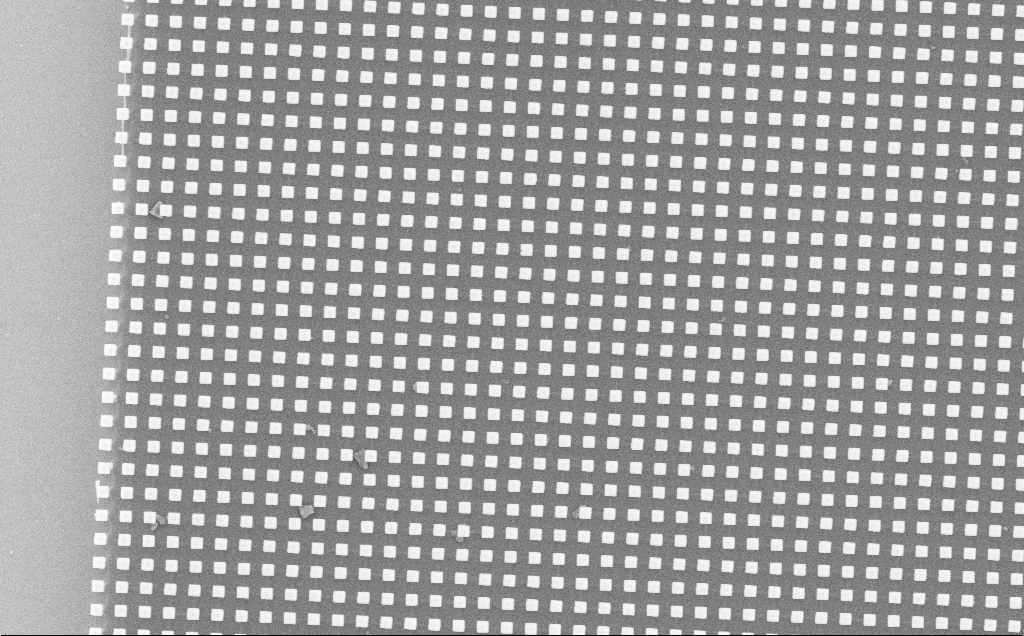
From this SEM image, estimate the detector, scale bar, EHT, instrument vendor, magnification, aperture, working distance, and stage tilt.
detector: SE2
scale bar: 10000 nm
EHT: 10 kV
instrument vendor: Zeiss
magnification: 2.22 K X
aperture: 30 µm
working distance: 16 mm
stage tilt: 0°